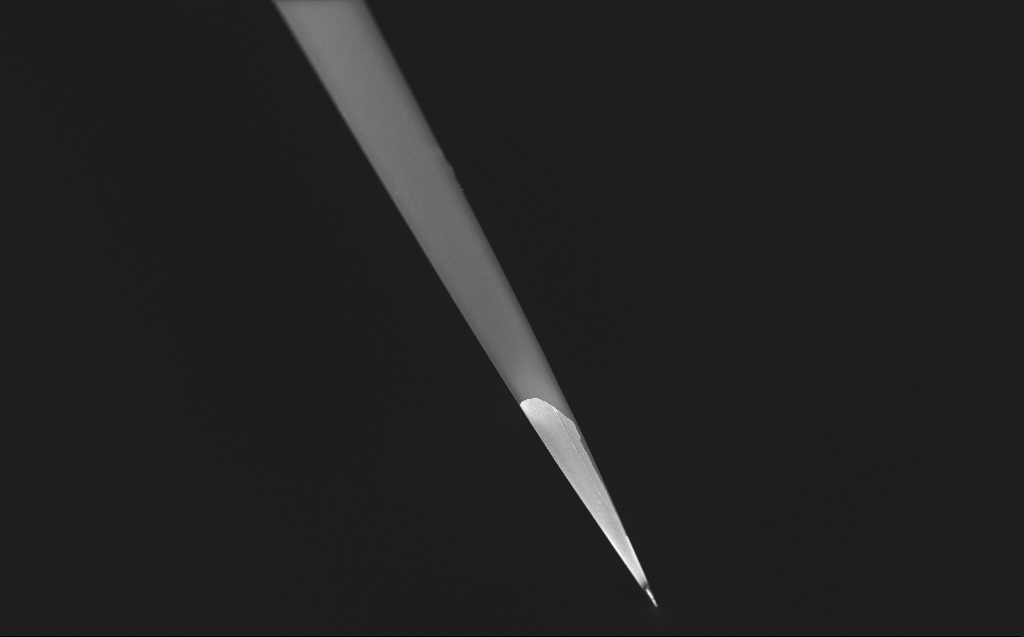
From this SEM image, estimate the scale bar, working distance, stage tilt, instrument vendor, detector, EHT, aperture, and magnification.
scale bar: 20000 nm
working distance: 4 mm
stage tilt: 45°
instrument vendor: Zeiss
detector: InLens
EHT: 0.8 kV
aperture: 30 µm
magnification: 1 K X